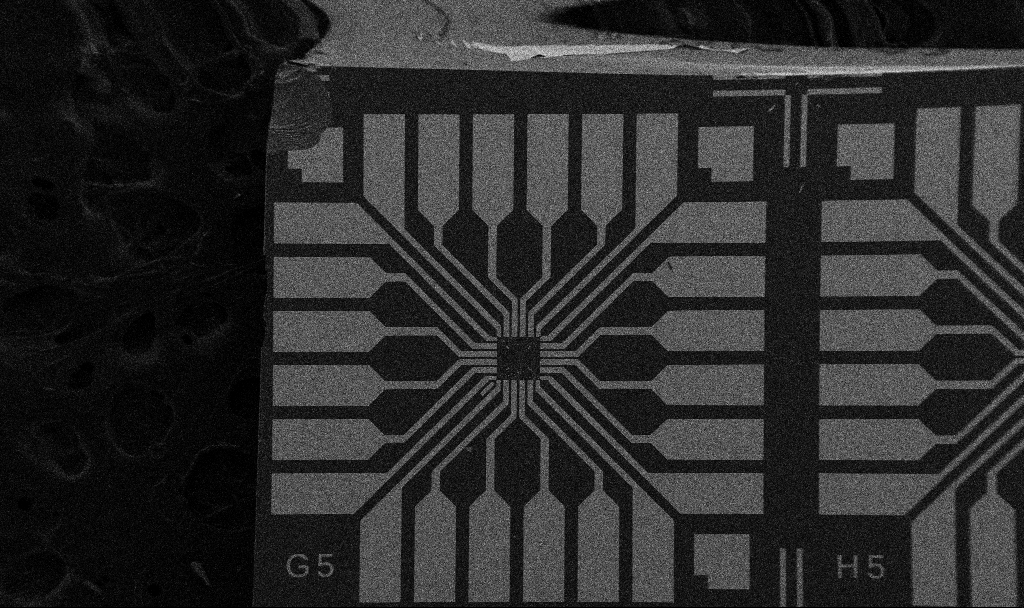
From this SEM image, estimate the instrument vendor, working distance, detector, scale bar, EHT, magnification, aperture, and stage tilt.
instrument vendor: Zeiss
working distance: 10.7 mm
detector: SE2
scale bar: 200000 nm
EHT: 5 kV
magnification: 0.1 K X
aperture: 30 µm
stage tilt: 0°